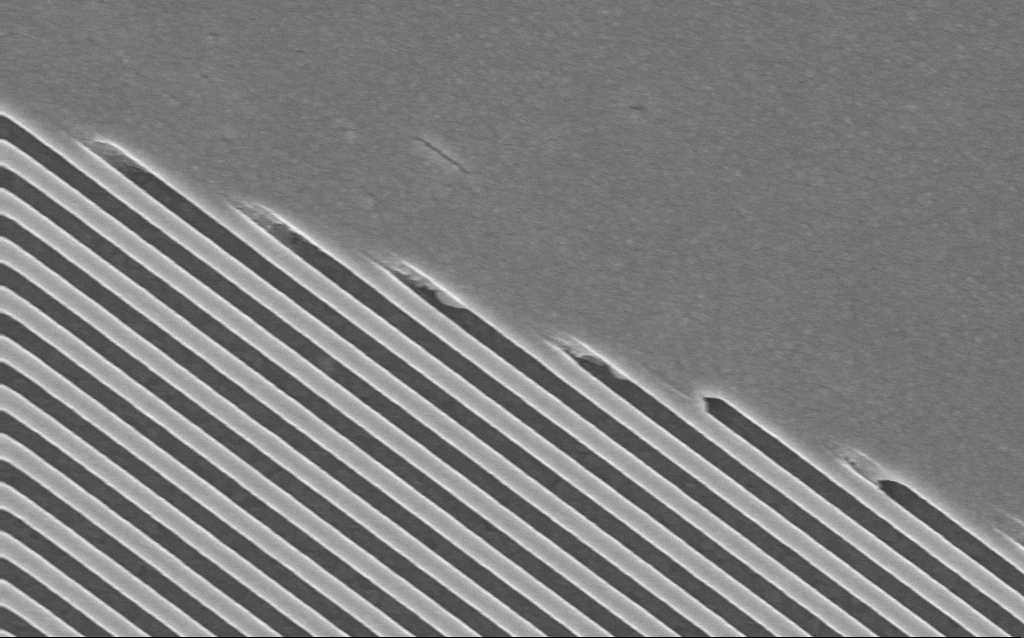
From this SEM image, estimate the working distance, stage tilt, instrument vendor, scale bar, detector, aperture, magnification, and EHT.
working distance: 3.9 mm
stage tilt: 0°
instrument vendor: Zeiss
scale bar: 1000 nm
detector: InLens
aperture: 30 µm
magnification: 36.29 K X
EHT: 10 kV